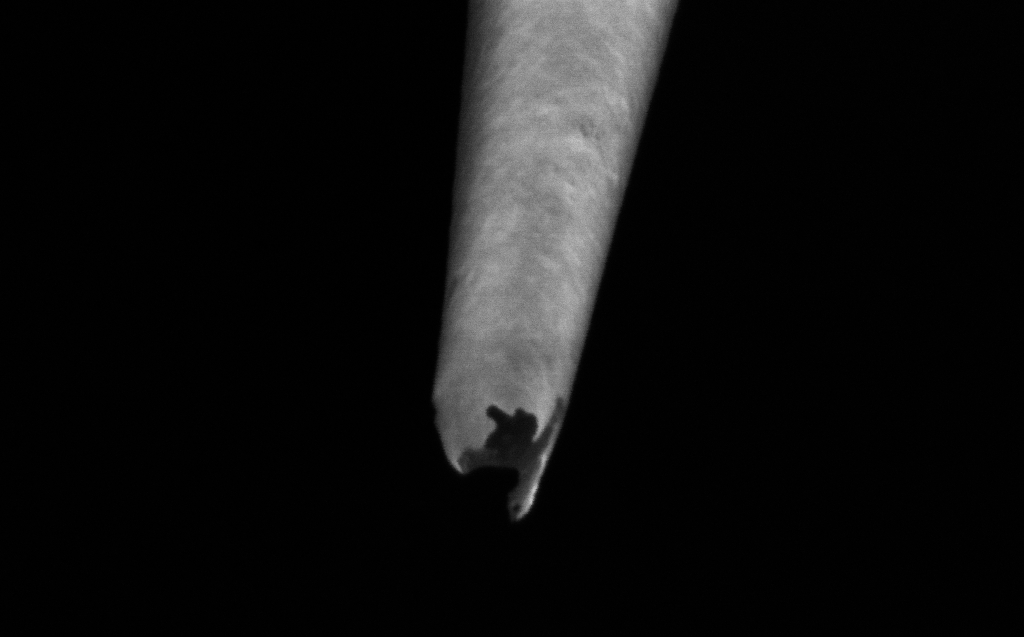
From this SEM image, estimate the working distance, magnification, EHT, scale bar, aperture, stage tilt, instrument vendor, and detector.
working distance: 4 mm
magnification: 250 K X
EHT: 2 kV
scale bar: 200 nm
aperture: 30 µm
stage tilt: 45°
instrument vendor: Zeiss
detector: InLens